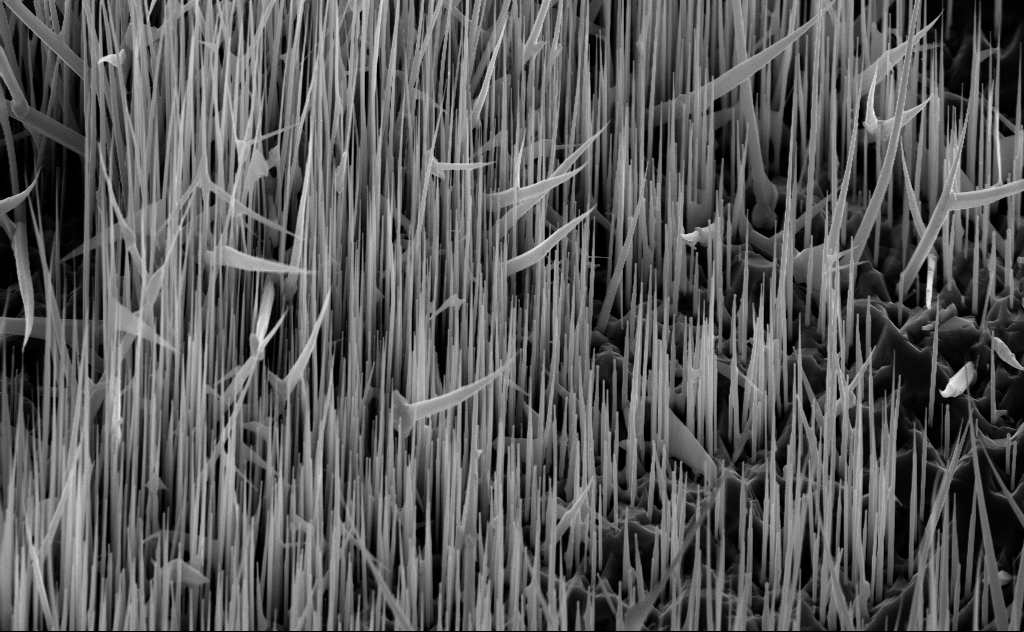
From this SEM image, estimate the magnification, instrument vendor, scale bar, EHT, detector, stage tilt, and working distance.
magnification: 14.64 K X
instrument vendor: Zeiss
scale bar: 2000 nm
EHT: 10 kV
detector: InLens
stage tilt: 45°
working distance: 7 mm